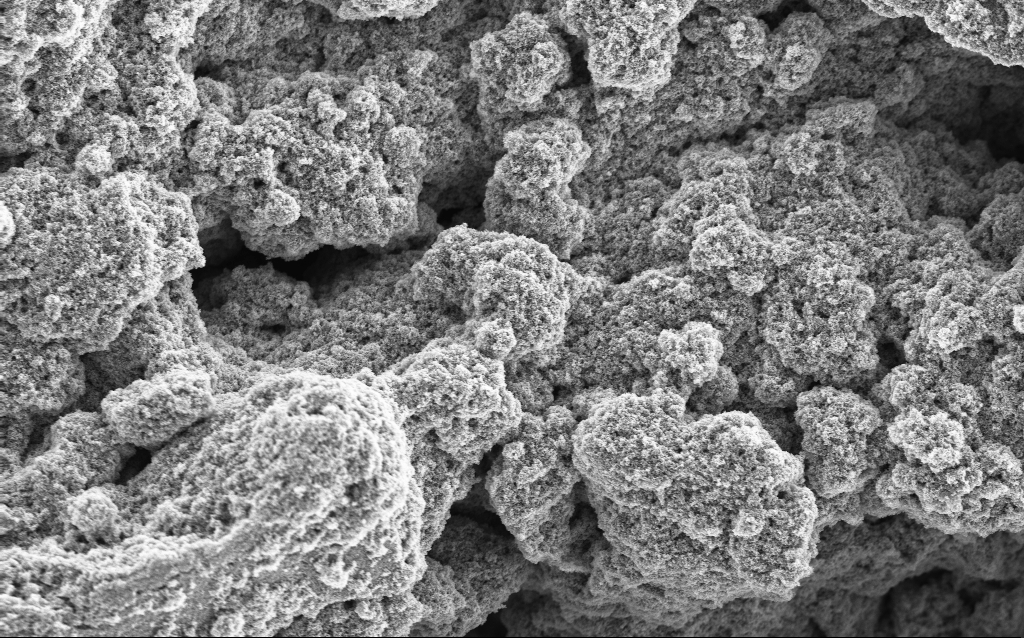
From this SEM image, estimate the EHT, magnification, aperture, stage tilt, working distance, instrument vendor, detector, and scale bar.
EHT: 5 kV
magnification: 6.4 K X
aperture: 30 µm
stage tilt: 0°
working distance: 4.5 mm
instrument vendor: Zeiss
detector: InLens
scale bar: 10000 nm